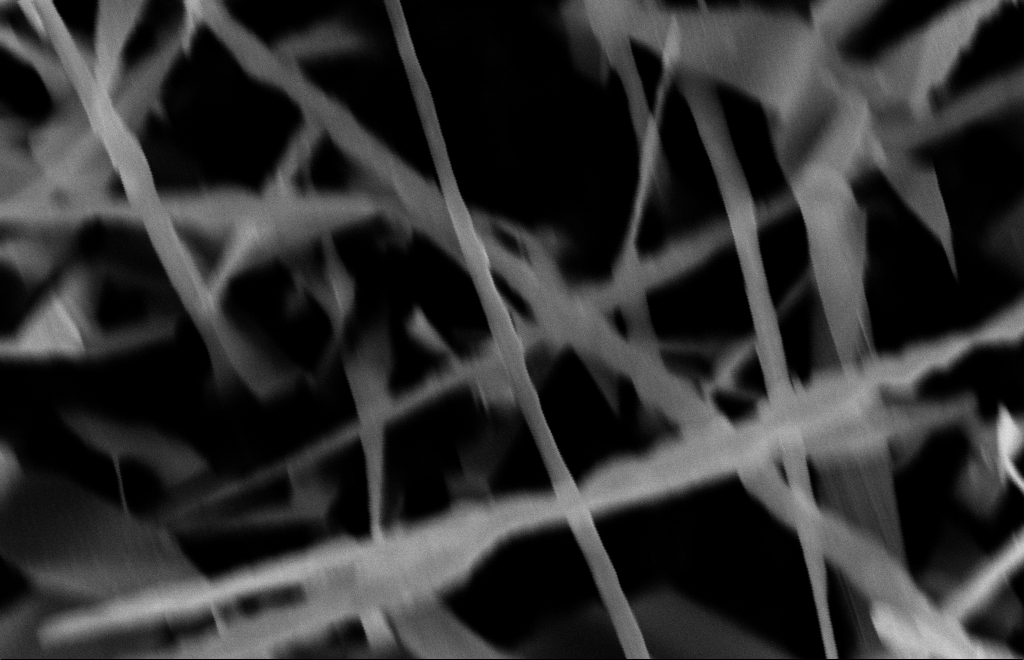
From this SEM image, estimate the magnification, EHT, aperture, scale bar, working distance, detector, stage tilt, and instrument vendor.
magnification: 80 K X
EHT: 10 kV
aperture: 30 µm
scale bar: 100 nm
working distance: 15 mm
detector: InLens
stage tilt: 0°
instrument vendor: Zeiss